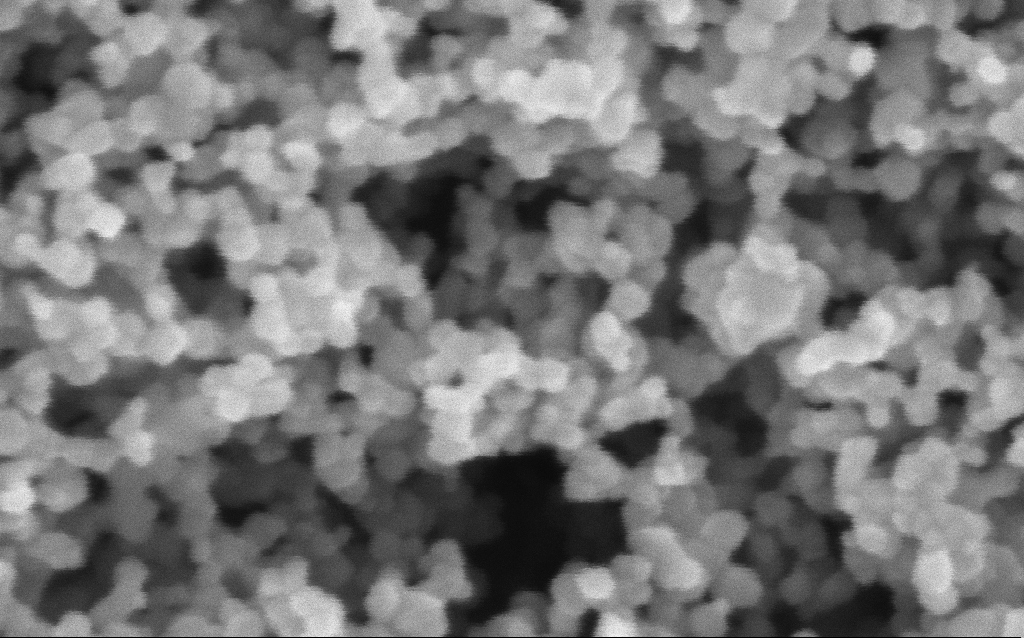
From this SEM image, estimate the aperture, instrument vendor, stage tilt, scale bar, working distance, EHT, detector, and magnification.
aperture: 30 µm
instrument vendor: Zeiss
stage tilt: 0°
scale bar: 100 nm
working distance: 7.5 mm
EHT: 3 kV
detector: InLens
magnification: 416 K X